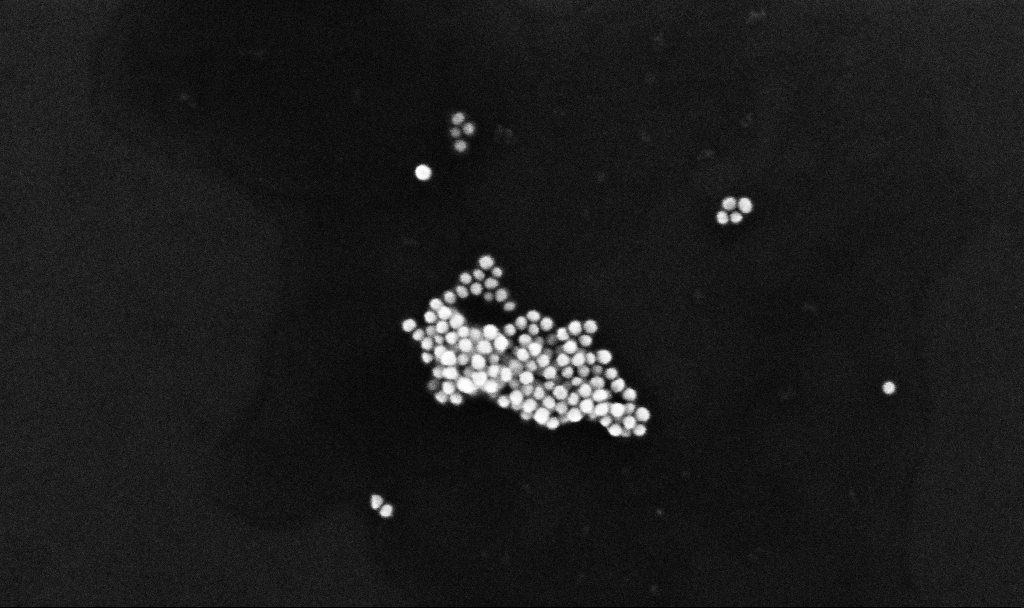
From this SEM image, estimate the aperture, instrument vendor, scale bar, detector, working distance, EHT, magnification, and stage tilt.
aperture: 30 µm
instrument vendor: Zeiss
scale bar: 200 nm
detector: InLens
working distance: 3.3 mm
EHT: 10 kV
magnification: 237.33 K X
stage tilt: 0°